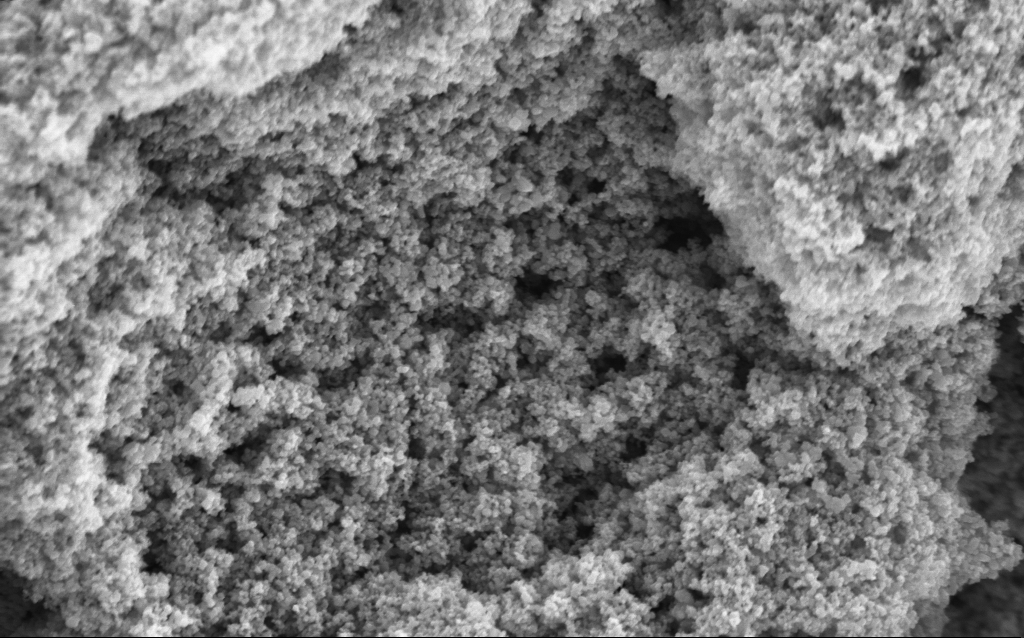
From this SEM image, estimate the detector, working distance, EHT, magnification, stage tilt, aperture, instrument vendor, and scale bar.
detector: InLens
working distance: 4.4 mm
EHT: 5 kV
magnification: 68.66 K X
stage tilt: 0°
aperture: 30 µm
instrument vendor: Zeiss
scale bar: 1000 nm